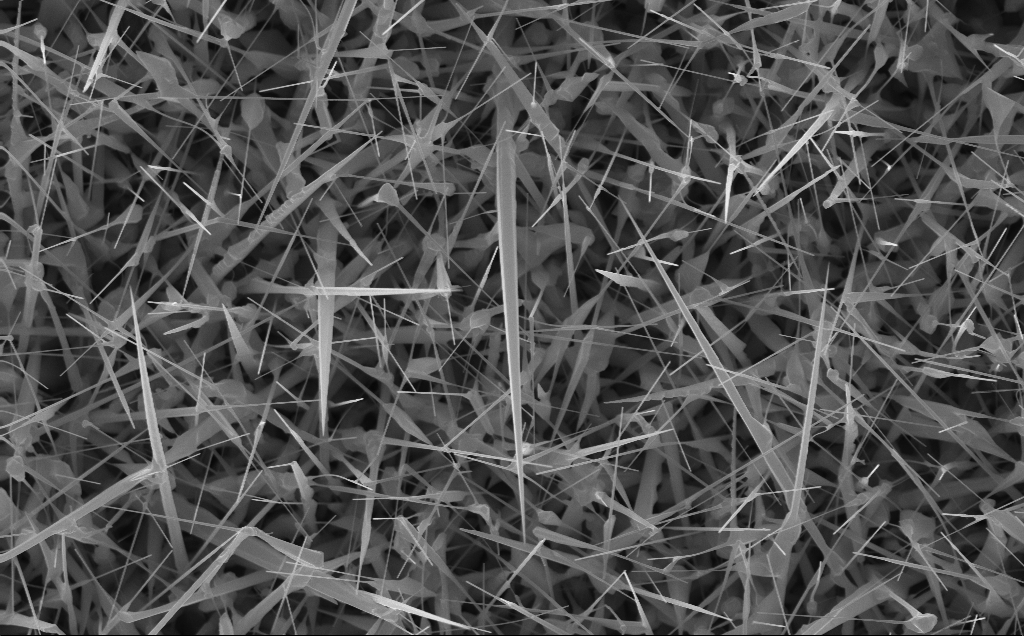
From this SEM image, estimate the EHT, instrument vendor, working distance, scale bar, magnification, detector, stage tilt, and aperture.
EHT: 10 kV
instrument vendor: Zeiss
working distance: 4 mm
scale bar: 2000 nm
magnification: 20 K X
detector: InLens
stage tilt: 0°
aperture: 30 µm